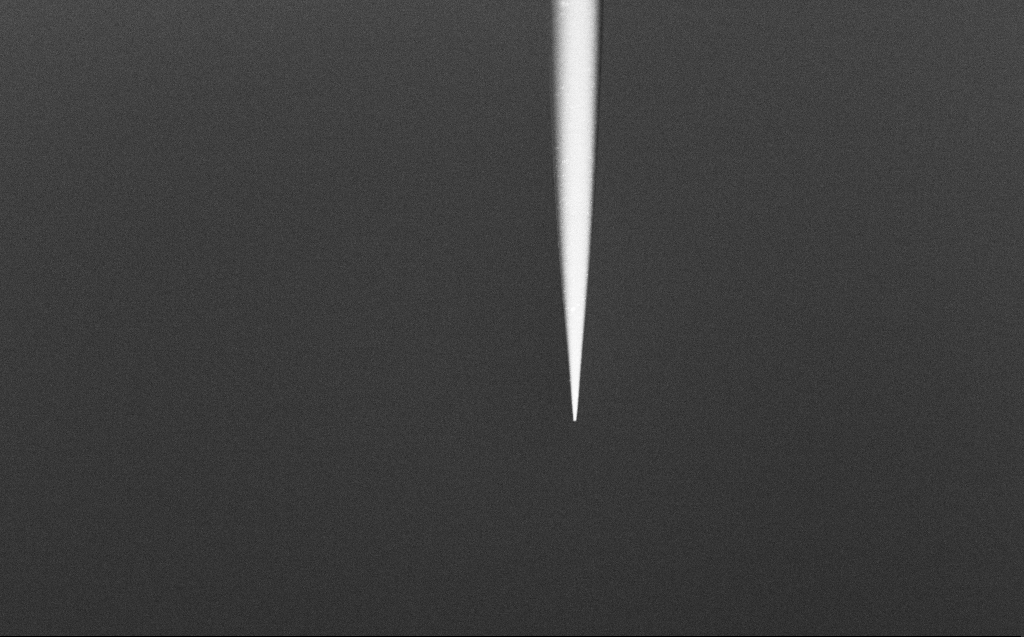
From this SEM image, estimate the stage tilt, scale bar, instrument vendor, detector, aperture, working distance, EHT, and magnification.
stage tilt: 45°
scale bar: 20000 nm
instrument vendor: Zeiss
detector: InLens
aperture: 30 µm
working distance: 4 mm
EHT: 4 kV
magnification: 1 K X